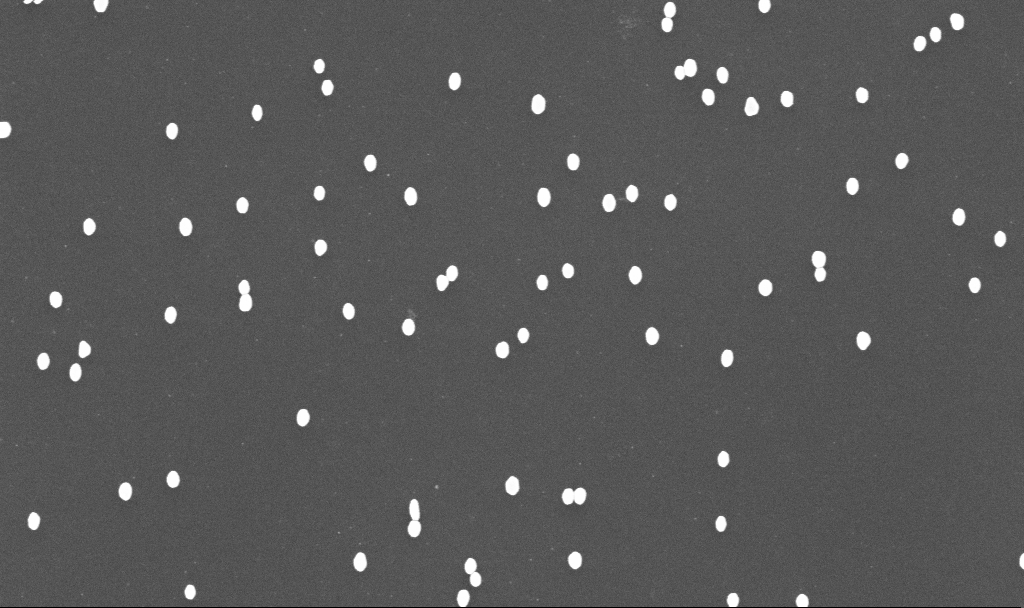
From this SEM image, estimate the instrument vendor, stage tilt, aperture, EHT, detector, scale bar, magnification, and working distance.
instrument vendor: Zeiss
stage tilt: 0°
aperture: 30 µm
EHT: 10 kV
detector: InLens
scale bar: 1000 nm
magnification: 70 K X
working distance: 3.4 mm